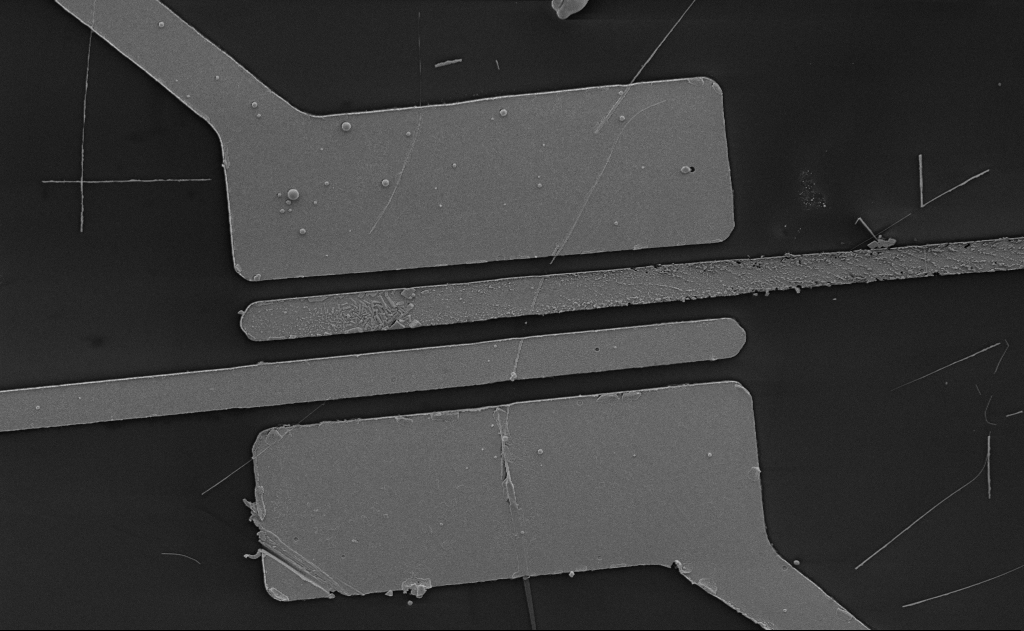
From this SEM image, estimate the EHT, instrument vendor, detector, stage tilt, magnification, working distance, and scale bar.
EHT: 5 kV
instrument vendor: Zeiss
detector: SE2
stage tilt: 0°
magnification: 6.06 K X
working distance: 15 mm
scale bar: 10000 nm